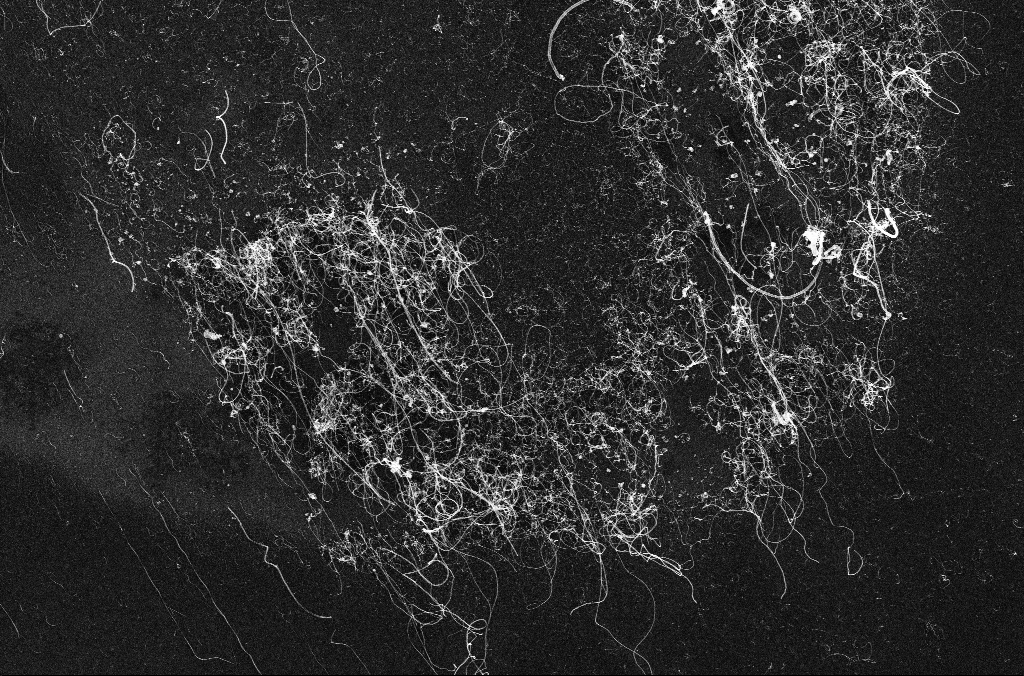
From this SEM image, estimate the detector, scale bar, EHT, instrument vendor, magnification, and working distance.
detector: InLens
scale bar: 2000 nm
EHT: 10 kV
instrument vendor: Zeiss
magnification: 10 K X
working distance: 3.3 mm